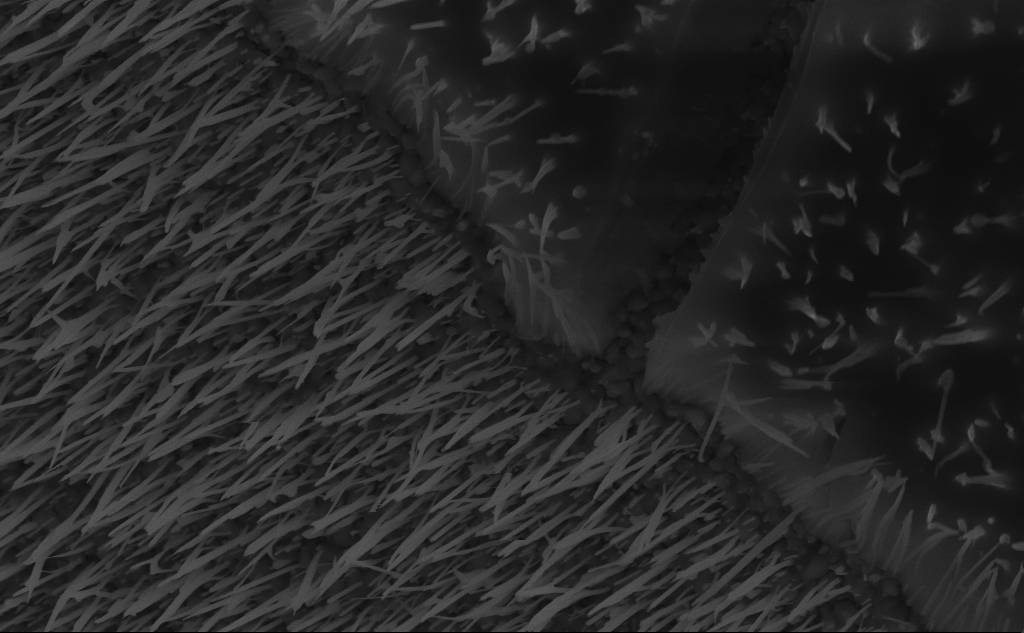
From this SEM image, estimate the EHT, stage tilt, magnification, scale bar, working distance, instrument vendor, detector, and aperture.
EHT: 10 kV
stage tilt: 45°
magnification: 35.6 K X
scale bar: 2000 nm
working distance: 6 mm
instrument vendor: Zeiss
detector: InLens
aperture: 30 µm